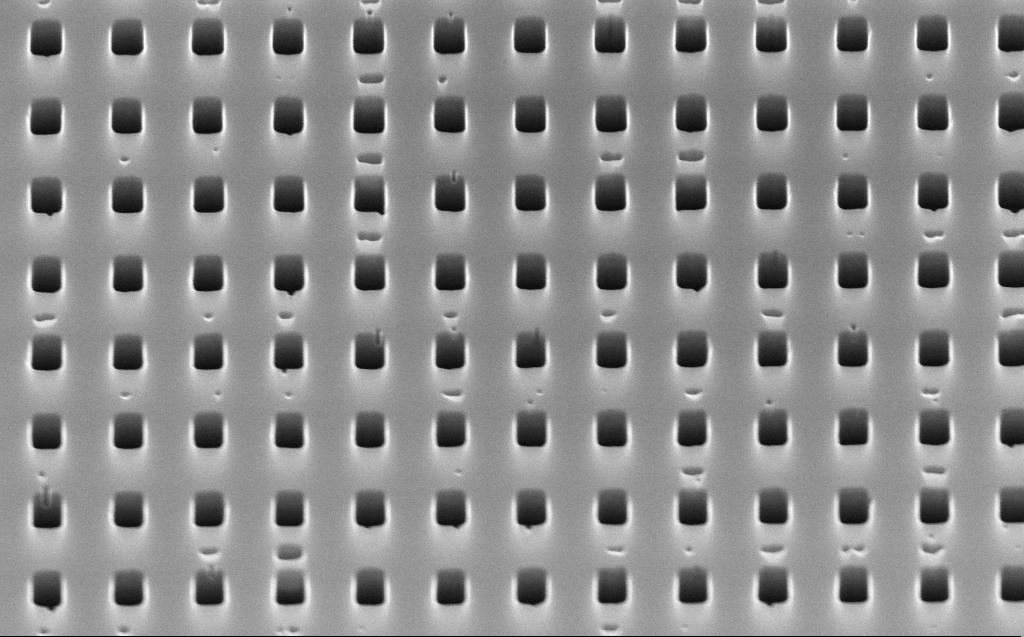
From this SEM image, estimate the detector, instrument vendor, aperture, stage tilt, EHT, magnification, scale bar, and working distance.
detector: InLens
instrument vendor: Zeiss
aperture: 30 µm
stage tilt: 45°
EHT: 10 kV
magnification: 60 K X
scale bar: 1000 nm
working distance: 4 mm